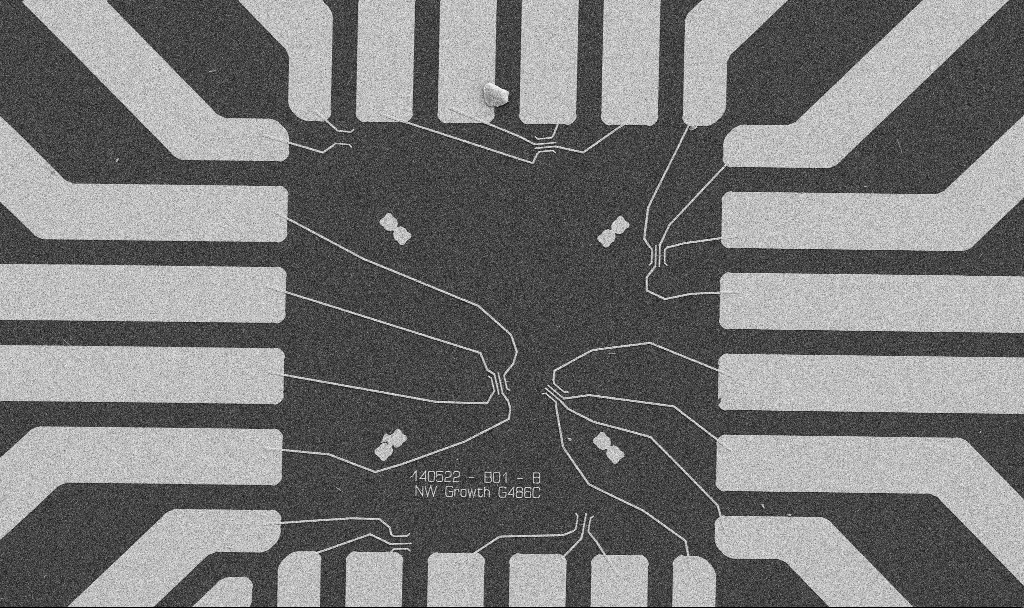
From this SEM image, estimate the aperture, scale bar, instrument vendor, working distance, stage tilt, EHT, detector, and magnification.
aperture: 30 µm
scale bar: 20000 nm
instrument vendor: Zeiss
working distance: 10.6 mm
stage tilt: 0°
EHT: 5 kV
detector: SE2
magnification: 1 K X